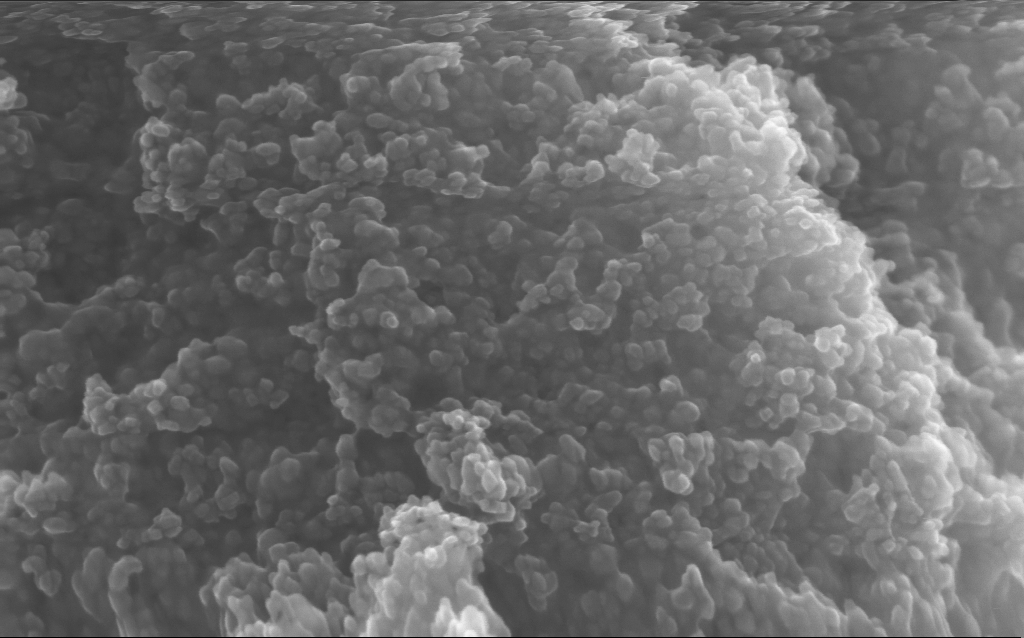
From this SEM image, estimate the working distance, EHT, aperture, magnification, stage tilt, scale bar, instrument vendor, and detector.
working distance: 2.7 mm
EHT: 10 kV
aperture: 30 µm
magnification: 211.33 K X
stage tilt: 0°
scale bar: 100 nm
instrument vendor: Zeiss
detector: InLens